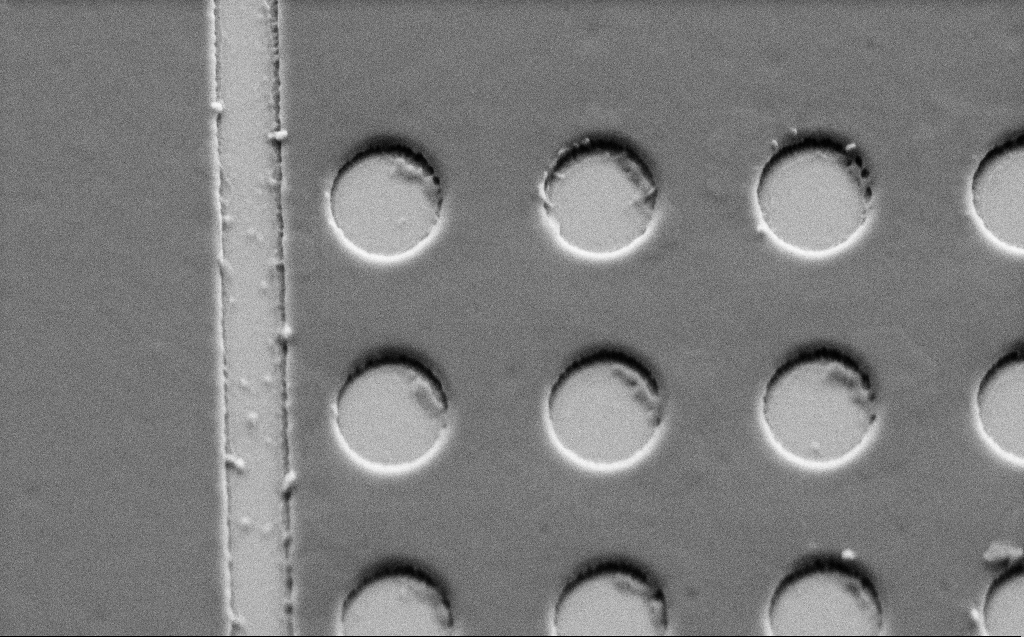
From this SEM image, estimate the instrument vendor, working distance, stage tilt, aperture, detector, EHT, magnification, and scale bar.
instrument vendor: Zeiss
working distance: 6 mm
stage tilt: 45°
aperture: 30 µm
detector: SE2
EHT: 3 kV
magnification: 19.78 K X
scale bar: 1000 nm